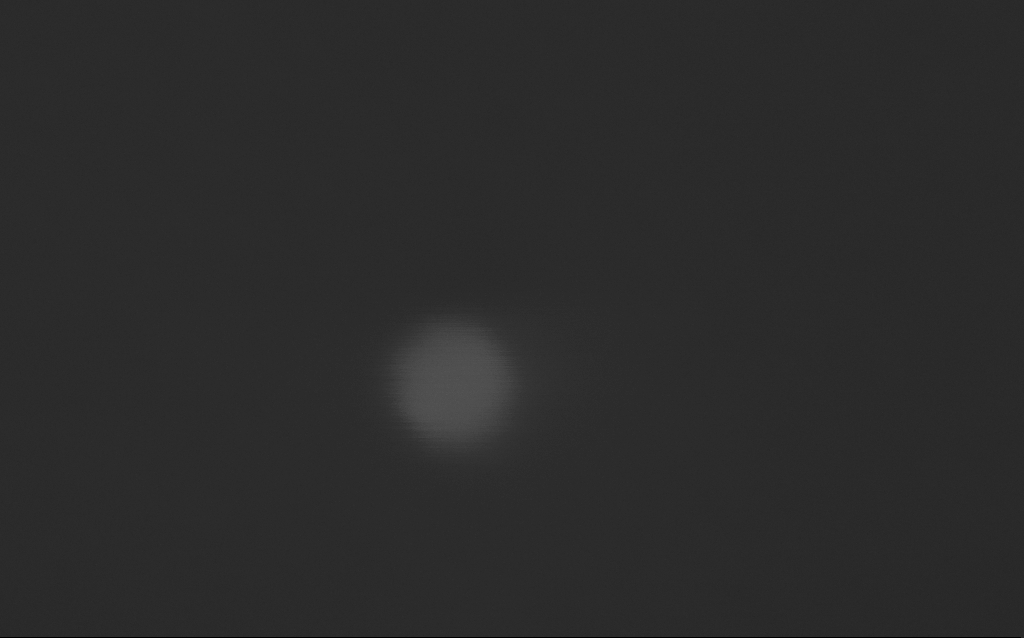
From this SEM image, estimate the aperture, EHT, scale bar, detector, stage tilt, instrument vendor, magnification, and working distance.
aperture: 30 µm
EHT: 3 kV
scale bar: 20 nm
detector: InLens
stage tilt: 0°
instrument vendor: Zeiss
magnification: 1824.59 K X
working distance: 3 mm